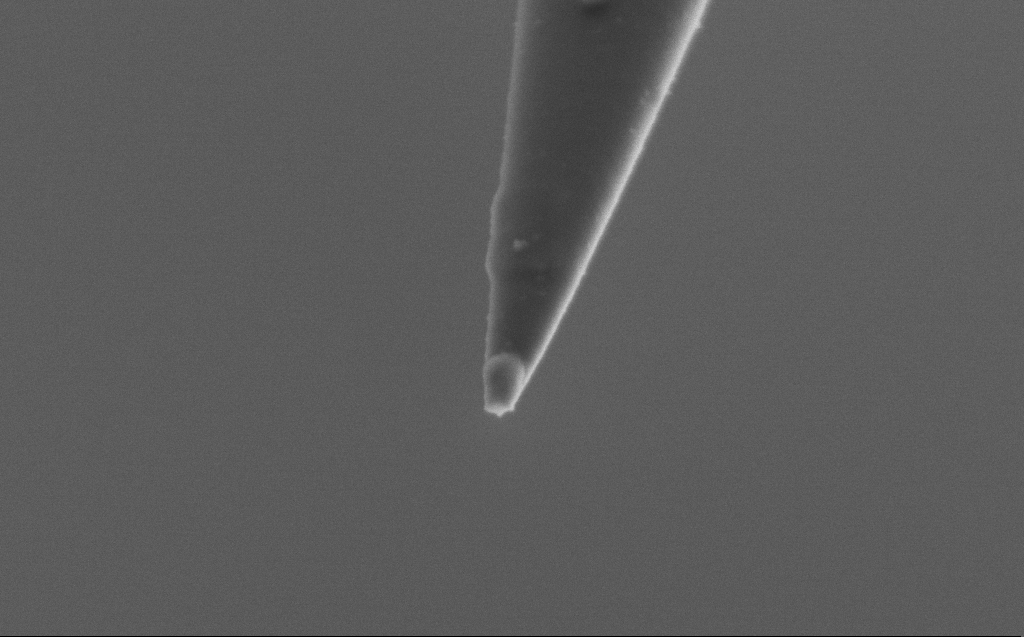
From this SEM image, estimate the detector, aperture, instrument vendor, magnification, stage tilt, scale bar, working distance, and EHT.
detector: SE2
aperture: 30 µm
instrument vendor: Zeiss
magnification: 100 K X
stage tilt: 45°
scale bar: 200 nm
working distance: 5 mm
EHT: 2 kV